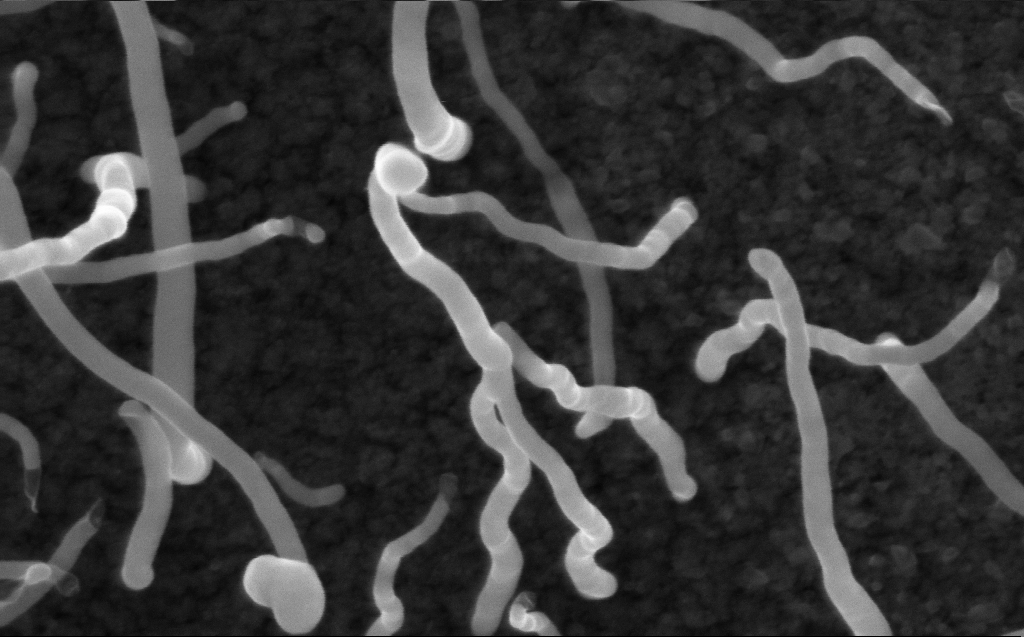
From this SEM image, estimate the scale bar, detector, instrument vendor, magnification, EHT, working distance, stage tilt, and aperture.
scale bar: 200 nm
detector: InLens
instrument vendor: Zeiss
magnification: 200 K X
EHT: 10 kV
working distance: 3 mm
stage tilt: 0°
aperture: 30 µm